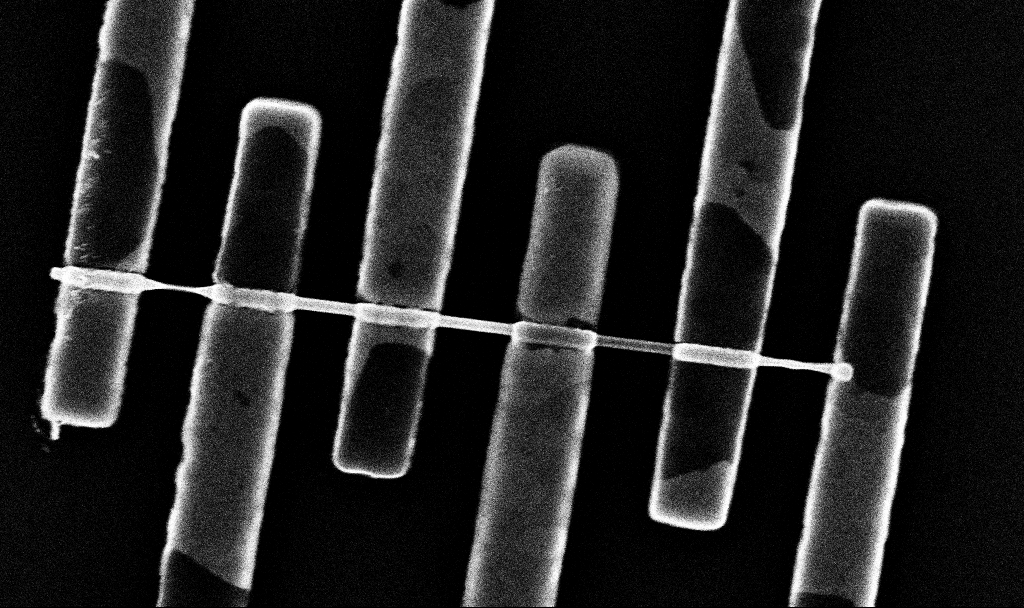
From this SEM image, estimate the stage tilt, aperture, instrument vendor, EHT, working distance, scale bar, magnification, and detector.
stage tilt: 0°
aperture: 30 µm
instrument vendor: Zeiss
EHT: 10 kV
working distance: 6.8 mm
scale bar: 1000 nm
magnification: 50.53 K X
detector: InLens